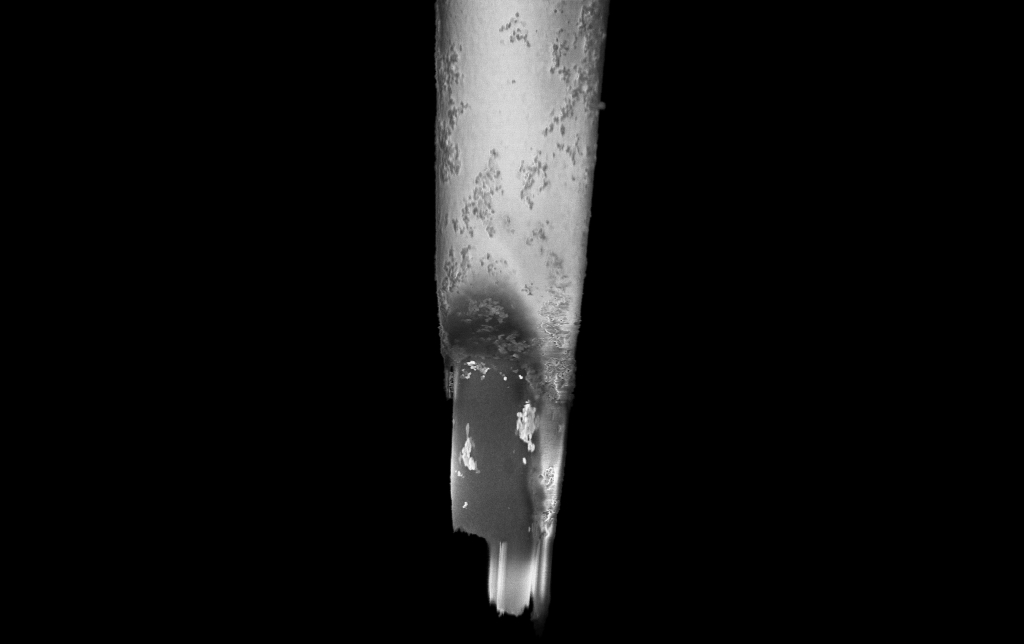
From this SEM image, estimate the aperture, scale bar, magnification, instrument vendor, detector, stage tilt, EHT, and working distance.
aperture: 30 µm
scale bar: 2000 nm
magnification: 25 K X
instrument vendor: Zeiss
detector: InLens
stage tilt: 0°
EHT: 2 kV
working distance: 6 mm